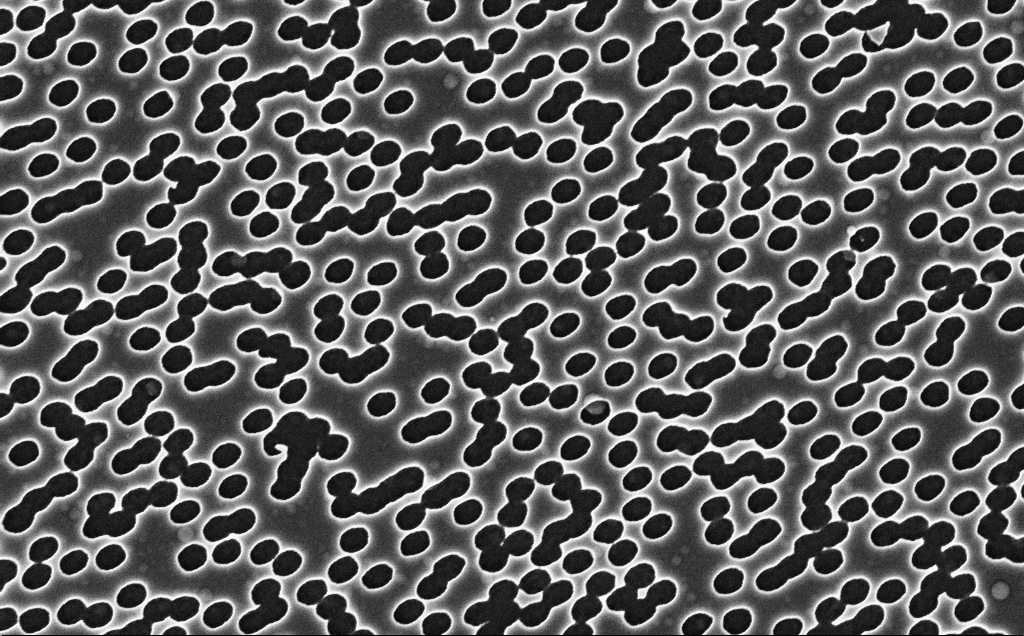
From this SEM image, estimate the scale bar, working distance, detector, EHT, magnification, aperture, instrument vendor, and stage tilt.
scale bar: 1000 nm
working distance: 2.5 mm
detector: InLens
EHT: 3 kV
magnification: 40 K X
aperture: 30 µm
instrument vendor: Zeiss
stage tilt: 0°